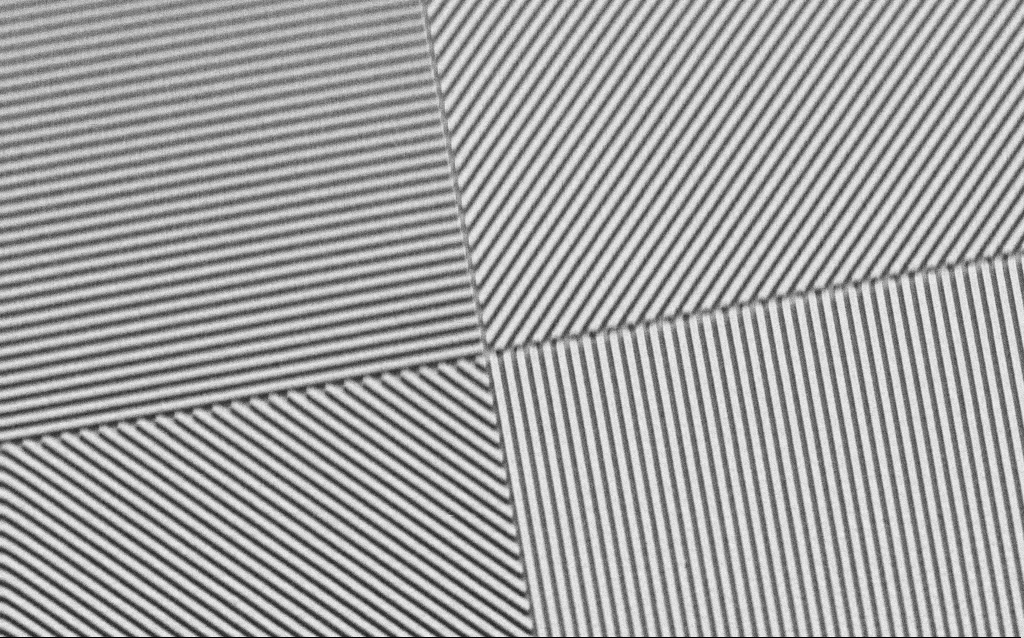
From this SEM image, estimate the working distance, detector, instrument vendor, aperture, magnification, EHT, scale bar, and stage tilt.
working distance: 6.2 mm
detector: SE2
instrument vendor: Zeiss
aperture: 30 µm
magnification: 12.43 K X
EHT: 3 kV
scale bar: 2000 nm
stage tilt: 45°